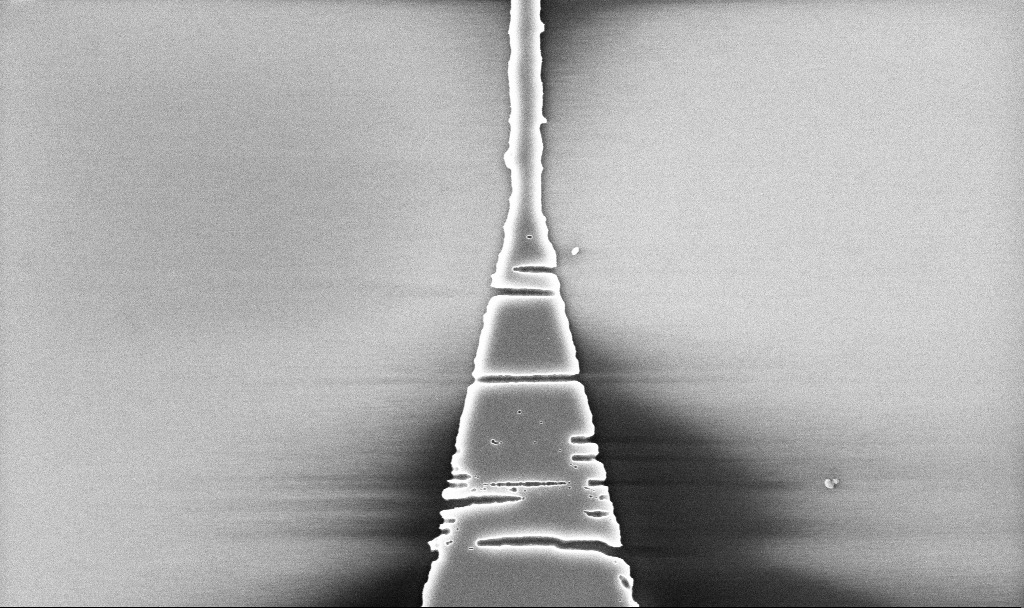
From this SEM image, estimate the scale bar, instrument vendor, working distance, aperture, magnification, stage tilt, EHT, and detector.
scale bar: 2000 nm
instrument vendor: Zeiss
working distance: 5.2 mm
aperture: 30 µm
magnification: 21.47 K X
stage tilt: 0°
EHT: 5 kV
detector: InLens